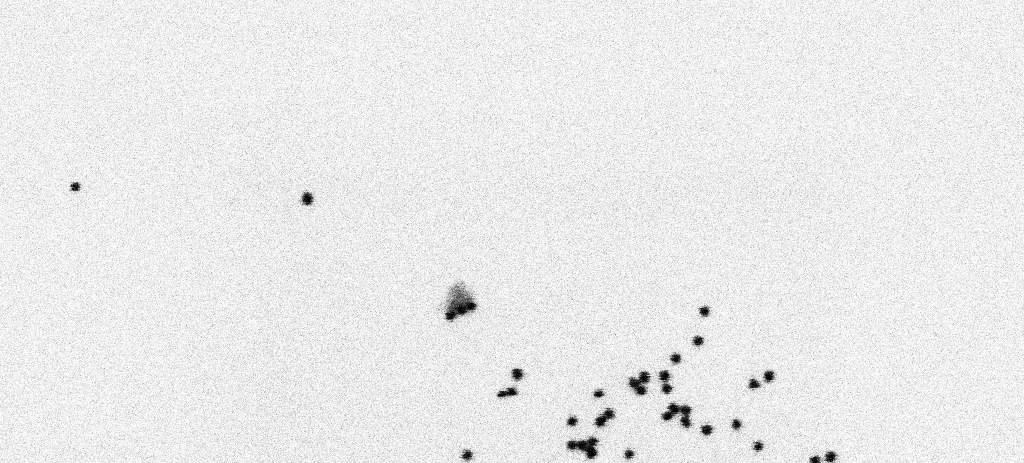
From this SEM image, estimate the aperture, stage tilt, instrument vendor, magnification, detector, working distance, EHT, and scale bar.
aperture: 30 µm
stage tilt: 0°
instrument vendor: Zeiss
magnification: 192.83 K X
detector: SE2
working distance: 6.5 mm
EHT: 2 kV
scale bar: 100 nm